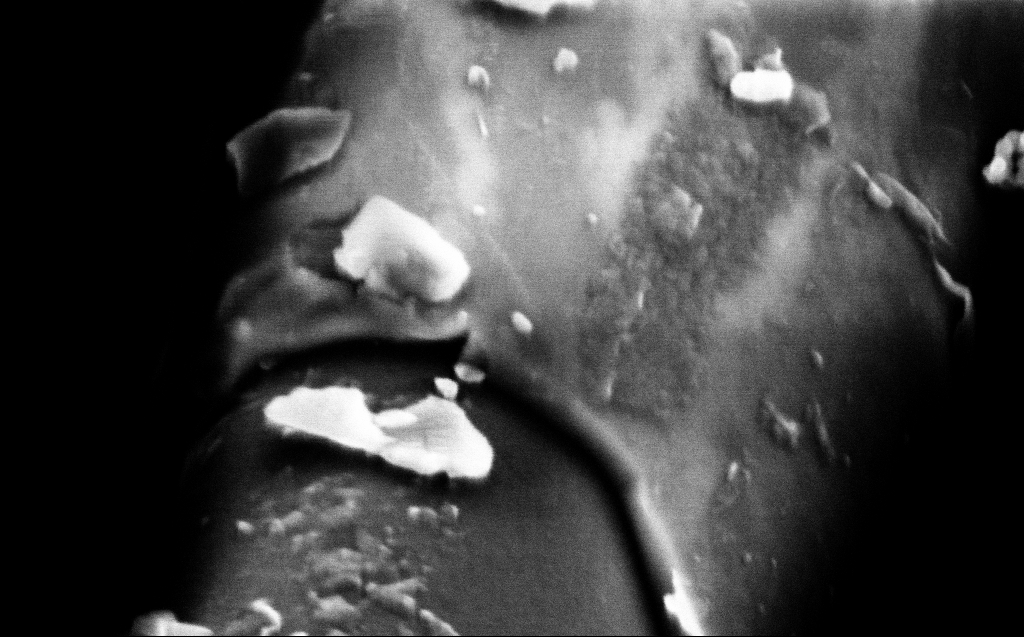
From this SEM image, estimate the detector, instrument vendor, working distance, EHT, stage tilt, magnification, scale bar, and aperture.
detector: InLens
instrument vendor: Zeiss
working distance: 5 mm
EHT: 2 kV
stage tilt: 45°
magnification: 200 K X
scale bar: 200 nm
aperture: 30 µm